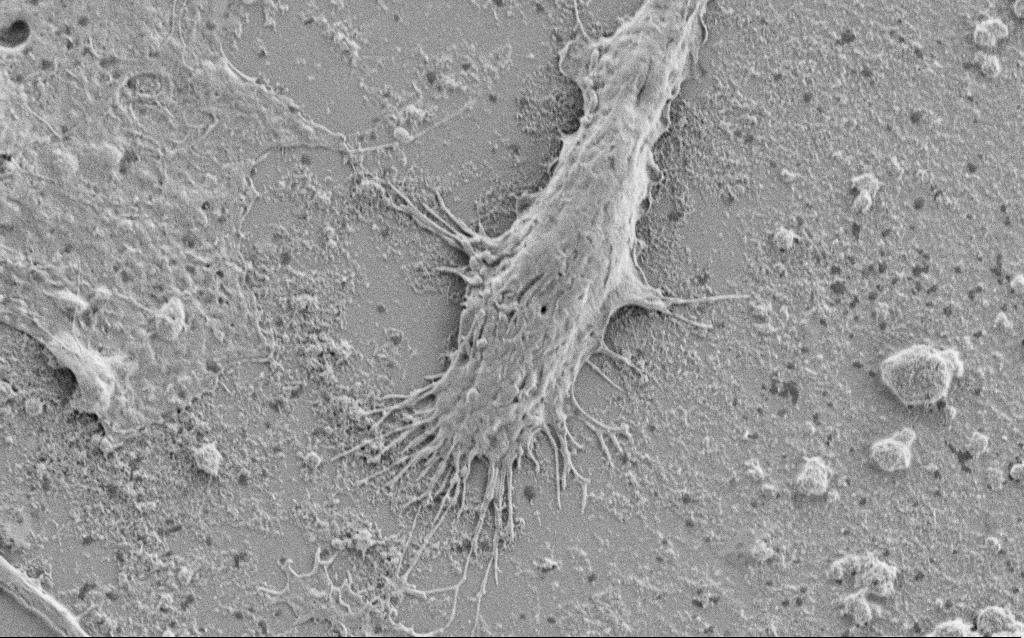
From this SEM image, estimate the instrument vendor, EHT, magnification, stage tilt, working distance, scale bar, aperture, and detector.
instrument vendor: Zeiss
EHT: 2 kV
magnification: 5 K X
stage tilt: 0°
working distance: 6.9 mm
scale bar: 10000 nm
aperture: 30 µm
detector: SE2